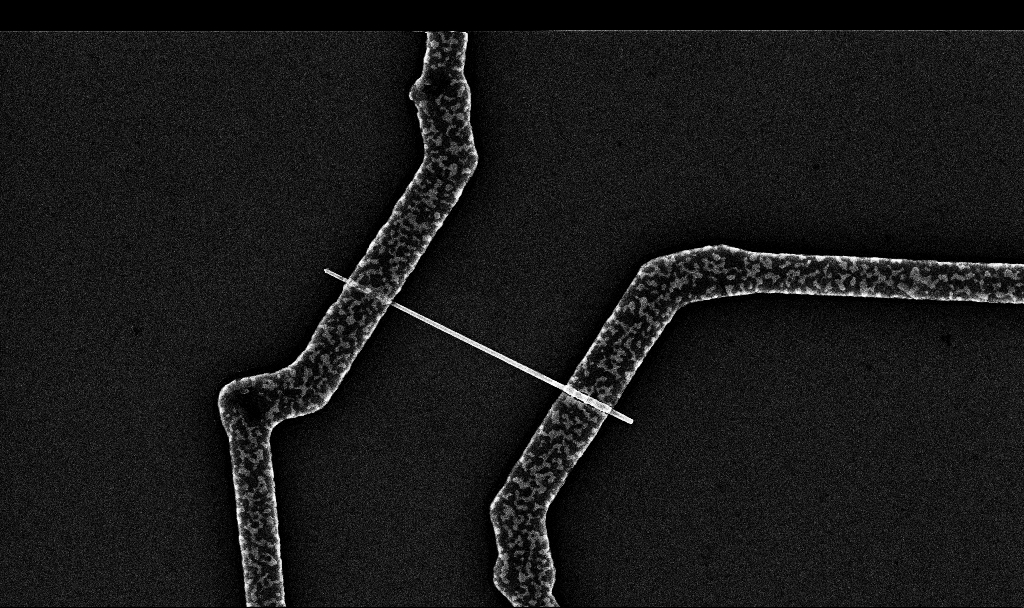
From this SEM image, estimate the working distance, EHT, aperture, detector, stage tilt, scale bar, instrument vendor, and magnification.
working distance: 6.7 mm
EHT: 10 kV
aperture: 30 µm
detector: InLens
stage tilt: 0°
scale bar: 1000 nm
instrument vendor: Zeiss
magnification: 20 K X